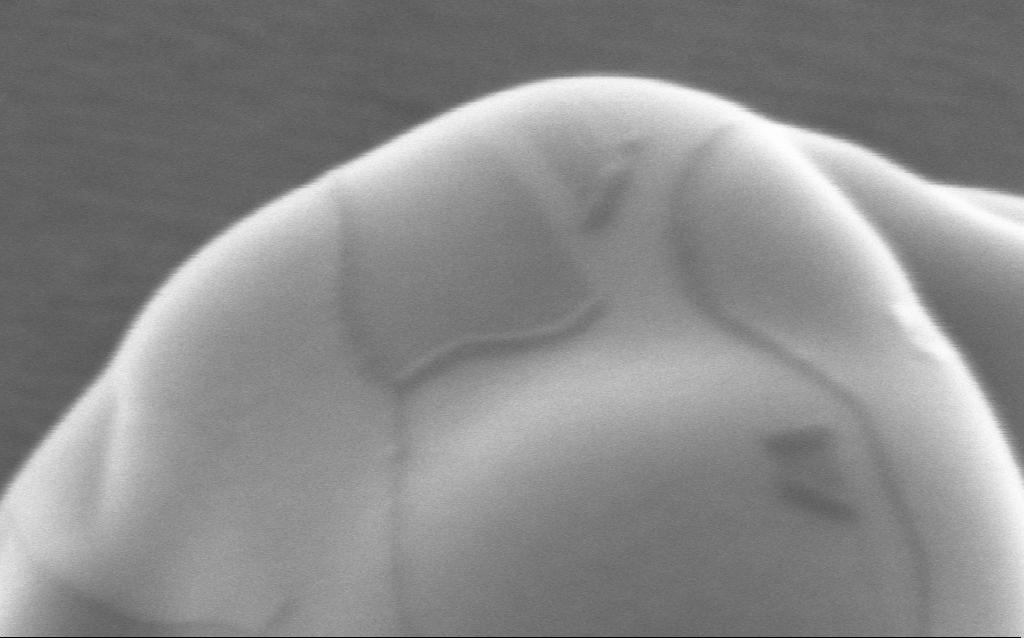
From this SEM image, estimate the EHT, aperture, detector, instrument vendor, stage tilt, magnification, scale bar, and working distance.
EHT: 5 kV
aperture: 30 µm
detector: InLens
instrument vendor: Zeiss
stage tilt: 0°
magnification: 595.06 K X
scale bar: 100 nm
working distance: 4 mm